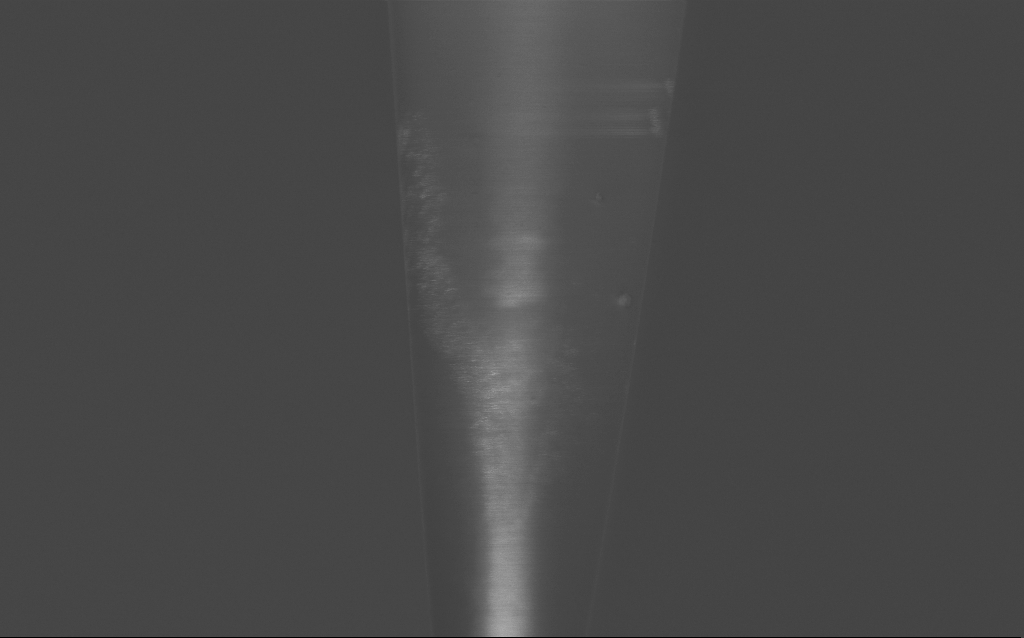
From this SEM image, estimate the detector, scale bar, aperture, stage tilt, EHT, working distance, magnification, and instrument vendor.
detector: InLens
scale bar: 2000 nm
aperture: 30 µm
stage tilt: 45°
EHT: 2 kV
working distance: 6 mm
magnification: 28.28 K X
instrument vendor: Zeiss